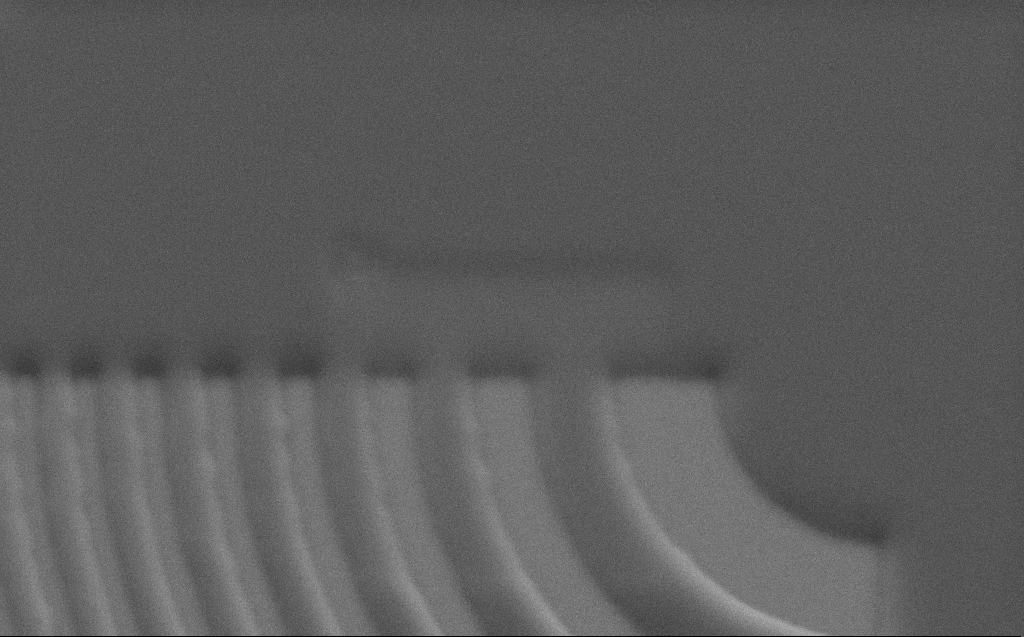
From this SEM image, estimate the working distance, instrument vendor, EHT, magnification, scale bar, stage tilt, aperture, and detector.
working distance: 5 mm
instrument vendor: Zeiss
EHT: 5 kV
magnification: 21.31 K X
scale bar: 2000 nm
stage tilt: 45°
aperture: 30 µm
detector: SE2